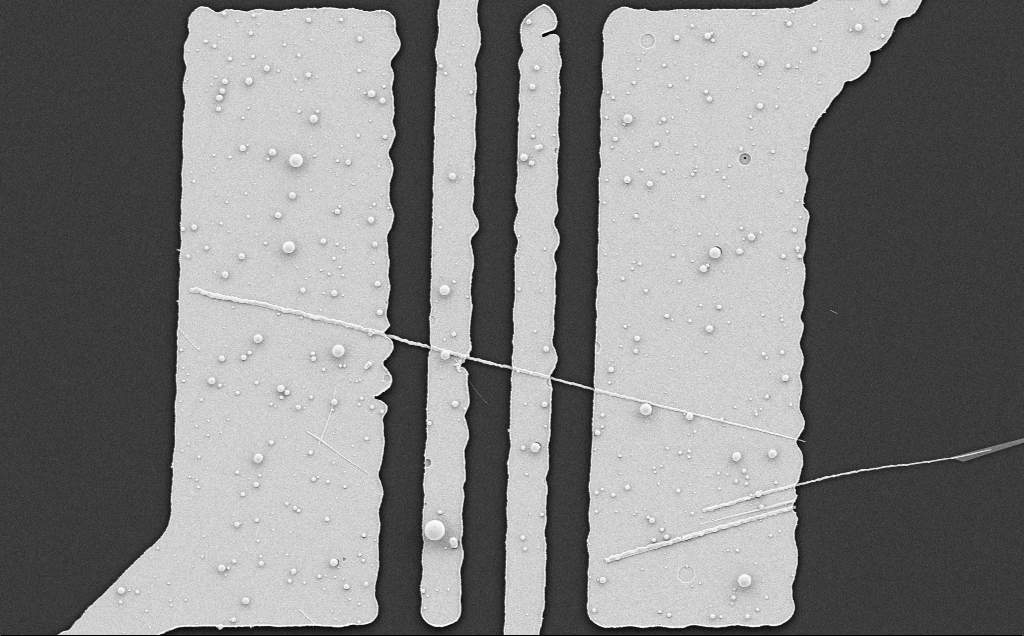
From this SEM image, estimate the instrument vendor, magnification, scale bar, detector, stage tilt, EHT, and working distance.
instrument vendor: Zeiss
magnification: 7.71 K X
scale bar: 2000 nm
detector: SE2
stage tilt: -0.7°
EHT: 5 kV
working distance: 8 mm